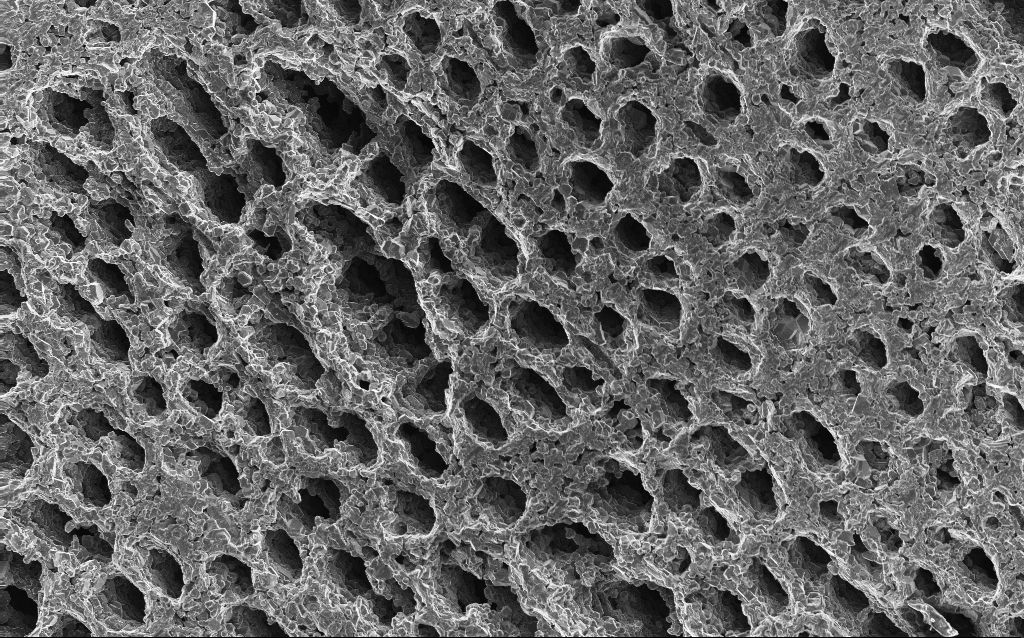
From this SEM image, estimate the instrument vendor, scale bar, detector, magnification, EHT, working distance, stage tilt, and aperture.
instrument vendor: Zeiss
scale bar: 100000 nm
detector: InLens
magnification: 0.5 K X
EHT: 10 kV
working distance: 3 mm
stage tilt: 0°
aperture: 30 µm